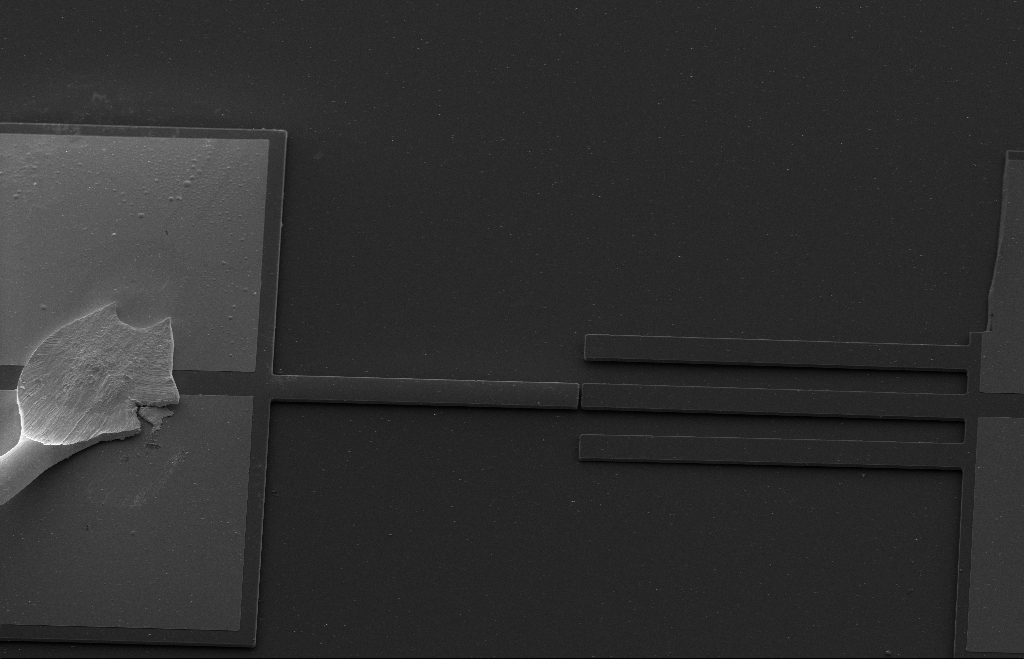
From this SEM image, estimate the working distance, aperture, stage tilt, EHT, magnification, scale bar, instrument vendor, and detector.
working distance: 9 mm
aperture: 20 µm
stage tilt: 33.1°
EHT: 10 kV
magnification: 0.57 K X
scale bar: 20000 nm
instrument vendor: Zeiss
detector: SE2